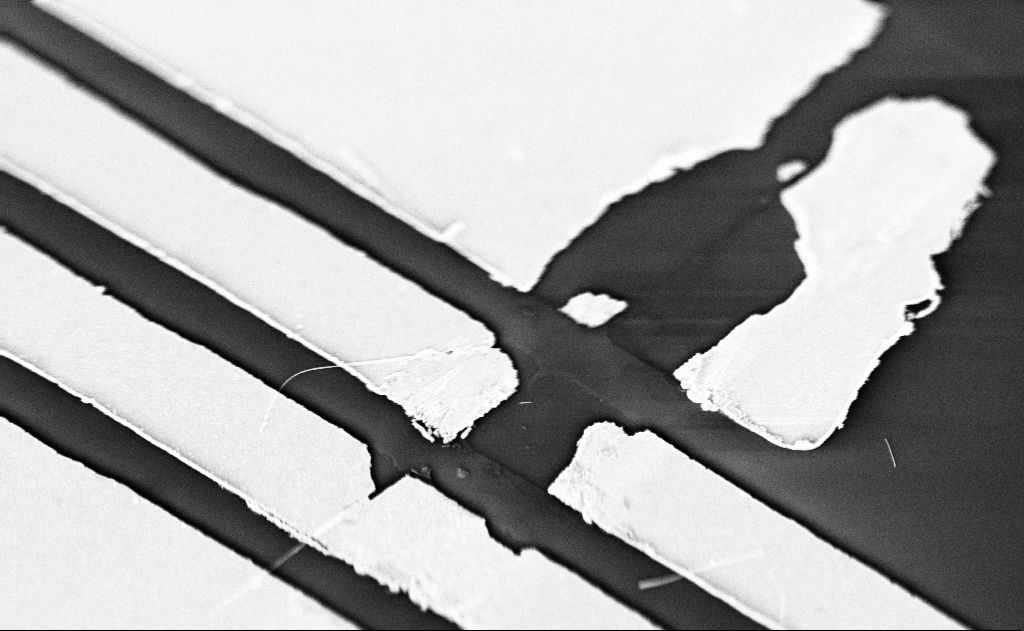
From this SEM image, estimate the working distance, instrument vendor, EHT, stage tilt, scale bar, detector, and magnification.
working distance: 20 mm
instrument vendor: Zeiss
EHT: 5 kV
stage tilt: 0°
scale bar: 2000 nm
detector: SE2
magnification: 14.44 K X